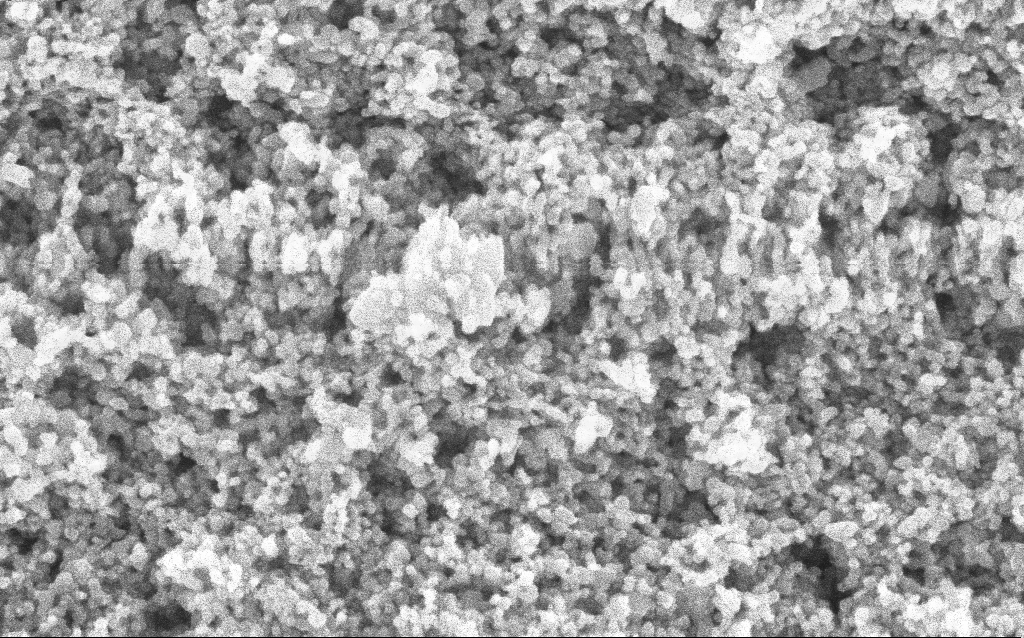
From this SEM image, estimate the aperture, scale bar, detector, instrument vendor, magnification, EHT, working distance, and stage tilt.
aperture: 30 µm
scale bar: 100 nm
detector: InLens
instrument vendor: Zeiss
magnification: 162.39 K X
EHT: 5 kV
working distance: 4.4 mm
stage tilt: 0°